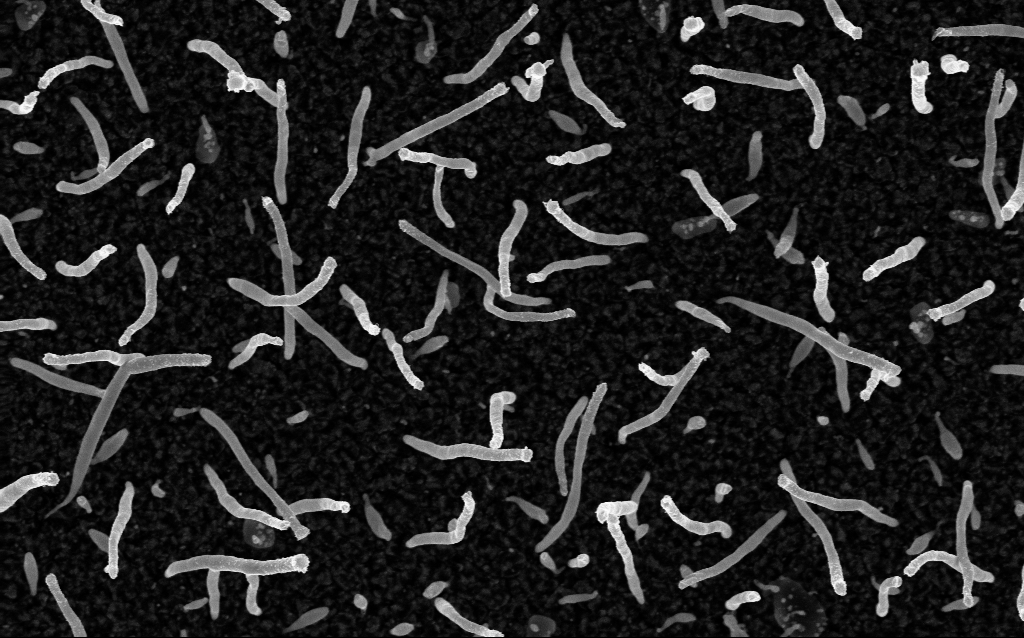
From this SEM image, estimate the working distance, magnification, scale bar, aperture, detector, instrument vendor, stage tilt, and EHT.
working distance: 1.8 mm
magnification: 50 K X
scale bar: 1000 nm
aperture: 30 µm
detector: InLens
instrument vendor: Zeiss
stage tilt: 0°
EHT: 5 kV